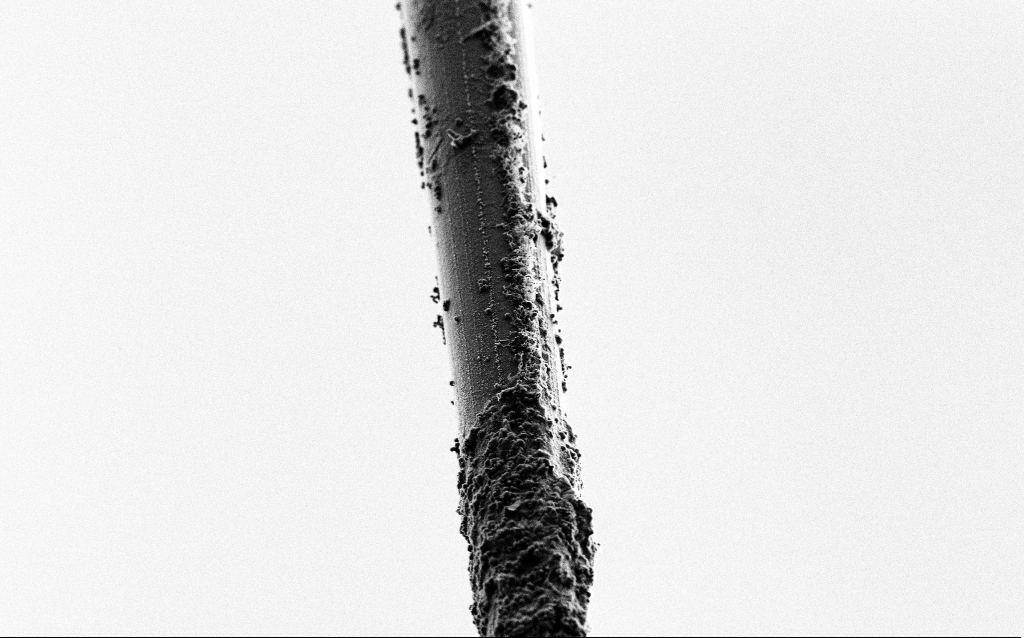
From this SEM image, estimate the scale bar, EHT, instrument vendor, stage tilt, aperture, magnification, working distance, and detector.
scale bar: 10000 nm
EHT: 5 kV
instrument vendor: Zeiss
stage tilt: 43.9°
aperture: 30 µm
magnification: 1.5 K X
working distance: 5.7 mm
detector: SE2